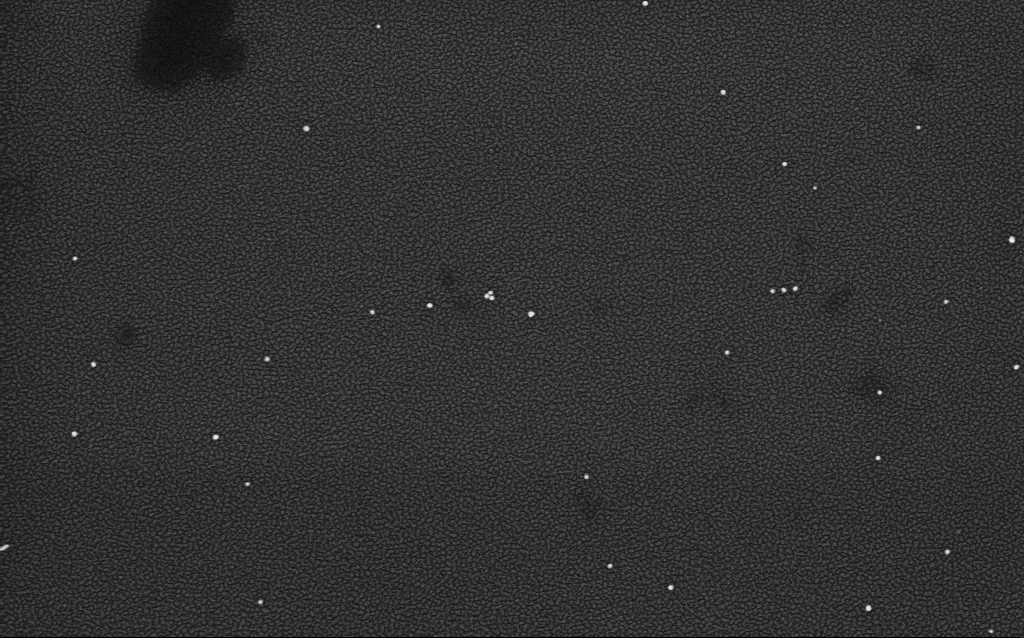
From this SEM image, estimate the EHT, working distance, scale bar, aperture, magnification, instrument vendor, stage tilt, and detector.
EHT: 4 kV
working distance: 2.1 mm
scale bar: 200 nm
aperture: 30 µm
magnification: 100 K X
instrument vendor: Zeiss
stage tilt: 0°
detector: InLens